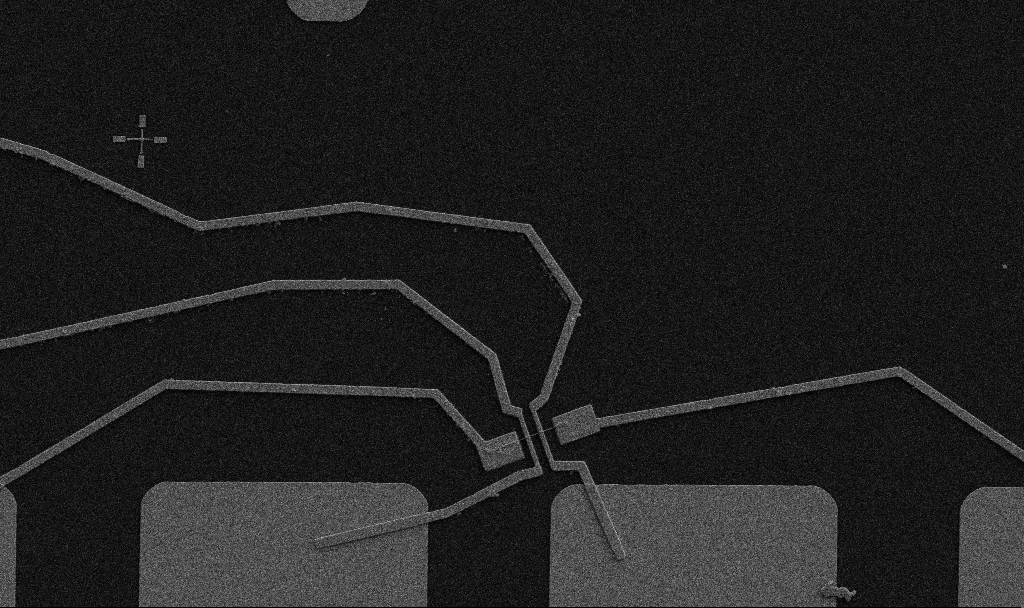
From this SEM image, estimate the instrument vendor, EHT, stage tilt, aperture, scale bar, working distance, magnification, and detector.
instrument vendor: Zeiss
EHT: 5 kV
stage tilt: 0°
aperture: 30 µm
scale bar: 10000 nm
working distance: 10.7 mm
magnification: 5 K X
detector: SE2